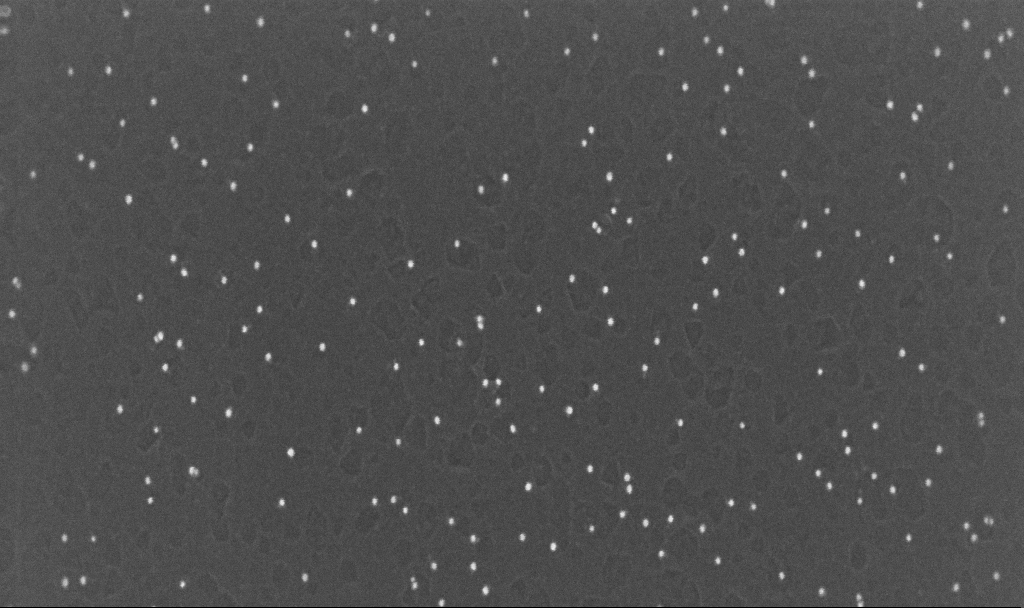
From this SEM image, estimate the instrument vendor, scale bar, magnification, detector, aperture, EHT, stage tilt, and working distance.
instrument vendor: Zeiss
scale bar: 200 nm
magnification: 200 K X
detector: InLens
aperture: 30 µm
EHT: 10 kV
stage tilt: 0°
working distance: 3.1 mm